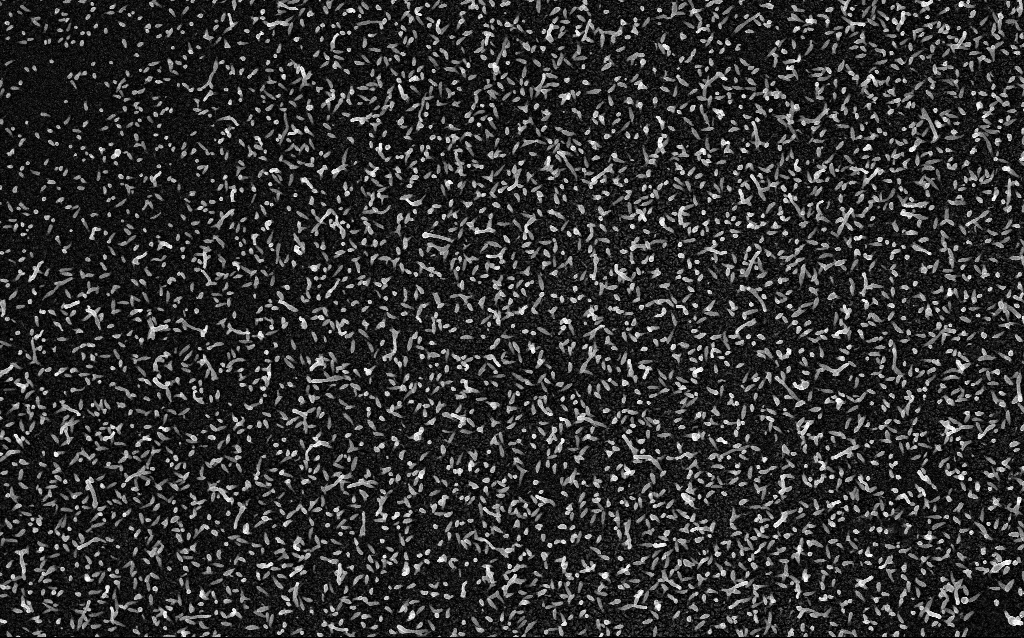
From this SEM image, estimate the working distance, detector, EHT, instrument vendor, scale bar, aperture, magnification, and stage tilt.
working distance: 2.1 mm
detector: InLens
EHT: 5 kV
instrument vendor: Zeiss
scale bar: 1000 nm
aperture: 30 µm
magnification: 20 K X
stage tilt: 0°